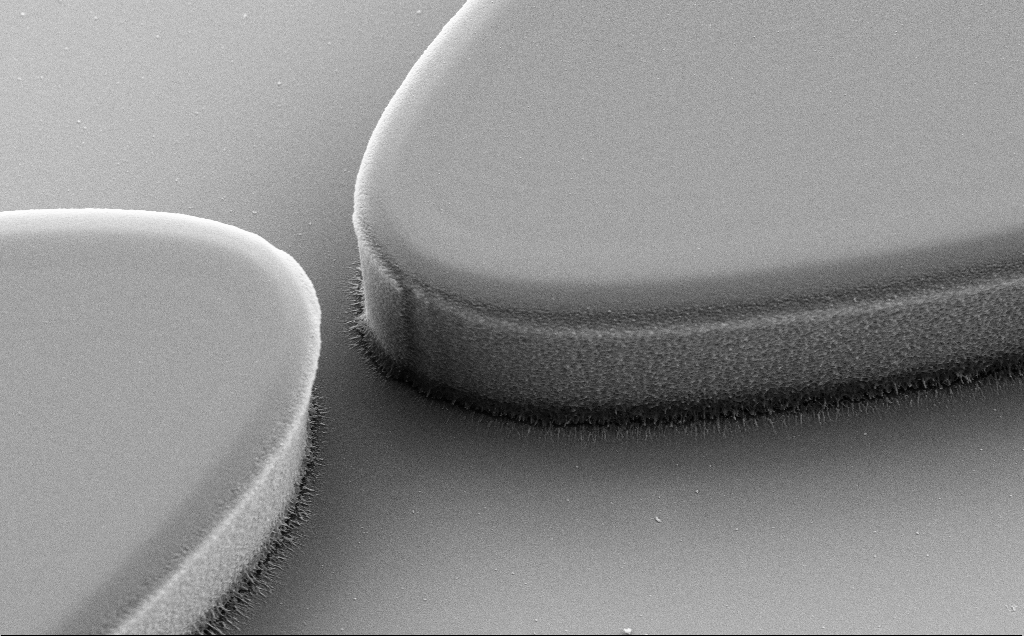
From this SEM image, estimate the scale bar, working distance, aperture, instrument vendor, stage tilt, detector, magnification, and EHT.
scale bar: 2000 nm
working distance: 8 mm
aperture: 30 µm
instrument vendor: Zeiss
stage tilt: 30°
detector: SE2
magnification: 8.08 K X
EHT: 5 kV